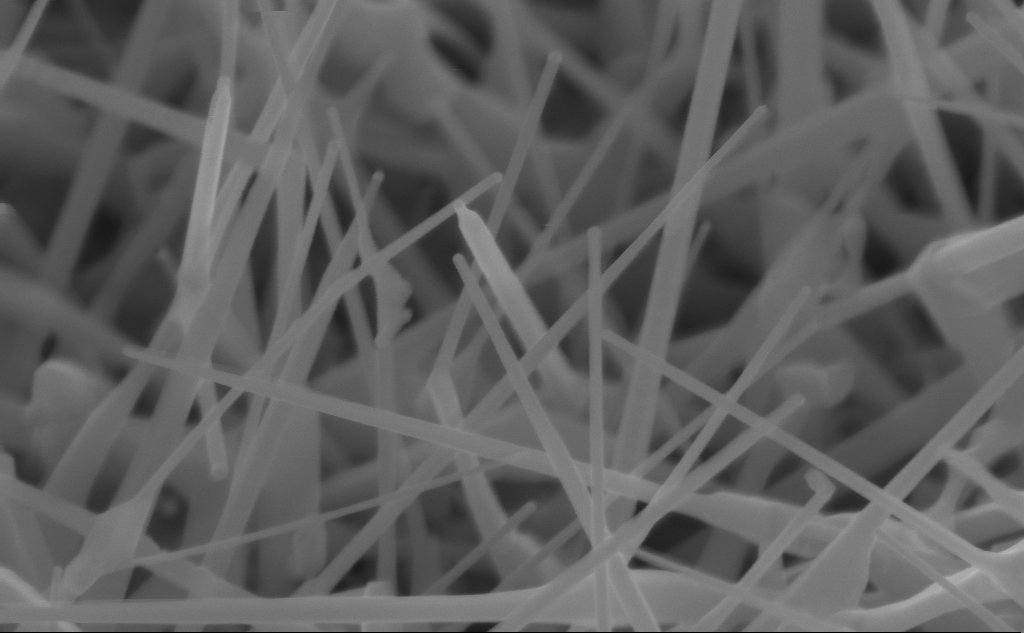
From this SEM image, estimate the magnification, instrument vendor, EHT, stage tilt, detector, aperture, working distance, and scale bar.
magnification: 150 K X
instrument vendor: Zeiss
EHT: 10 kV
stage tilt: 45°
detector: InLens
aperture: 30 µm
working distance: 4 mm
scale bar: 200 nm